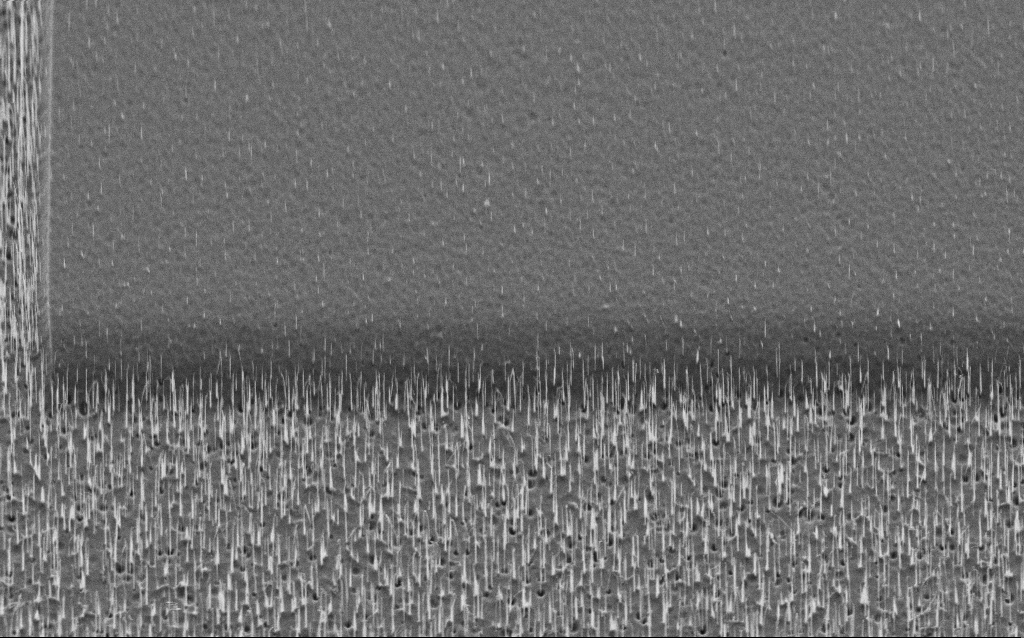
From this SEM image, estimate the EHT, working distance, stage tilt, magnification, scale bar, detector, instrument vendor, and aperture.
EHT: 5 kV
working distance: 8 mm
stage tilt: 45°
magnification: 5.4 K X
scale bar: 10000 nm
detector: SE2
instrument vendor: Zeiss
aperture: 30 µm